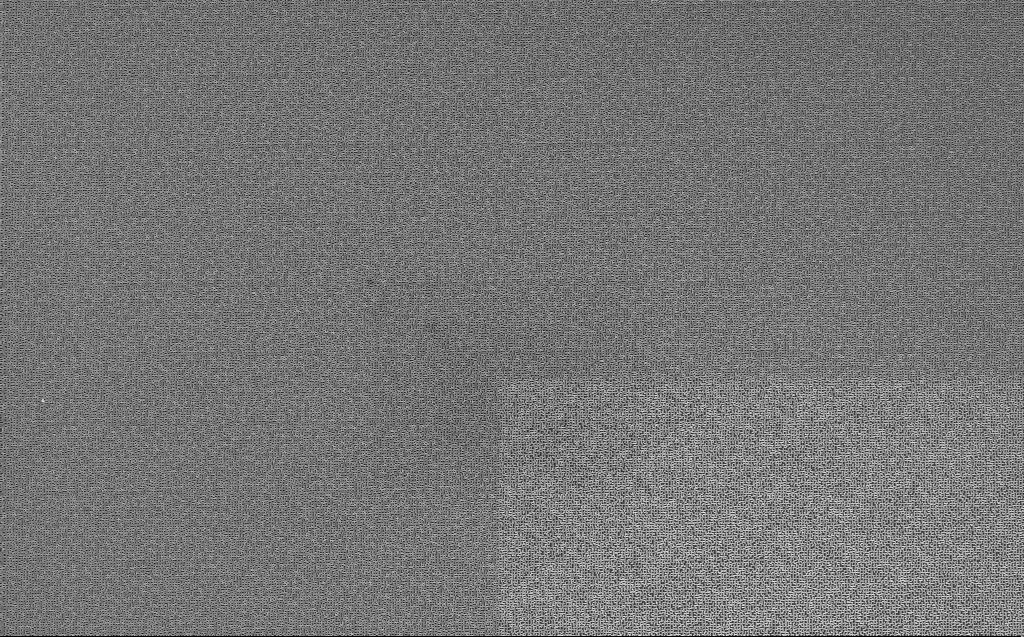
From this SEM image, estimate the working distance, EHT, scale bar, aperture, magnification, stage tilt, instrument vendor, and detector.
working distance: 7 mm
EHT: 5 kV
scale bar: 10000 nm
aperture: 30 µm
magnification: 2.47 K X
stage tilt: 0°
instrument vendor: Zeiss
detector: InLens